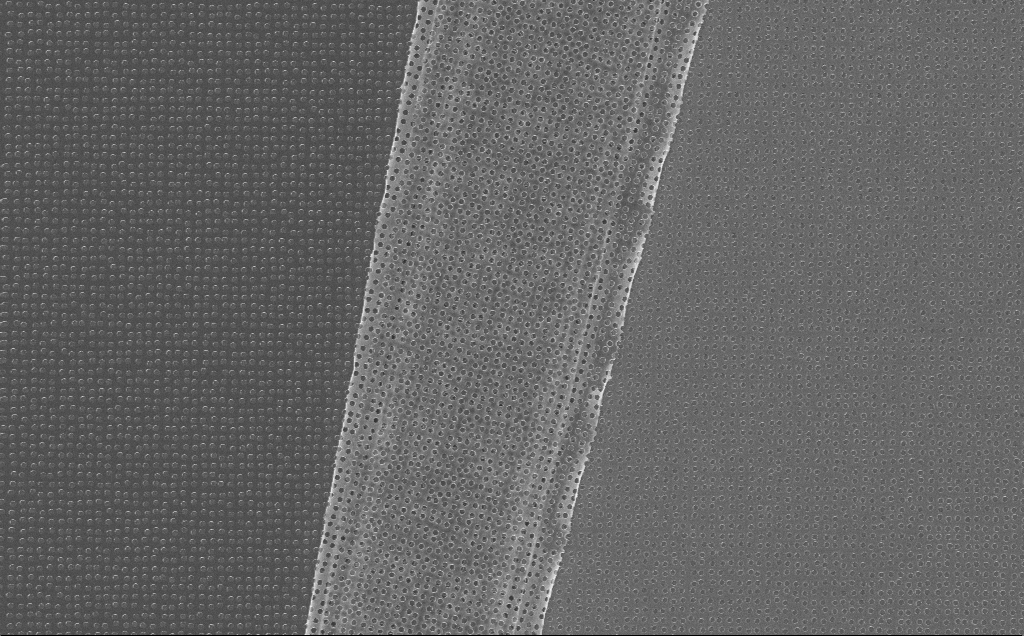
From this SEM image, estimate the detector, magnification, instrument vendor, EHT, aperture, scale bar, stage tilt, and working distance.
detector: InLens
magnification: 8.7 K X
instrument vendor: Zeiss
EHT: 10 kV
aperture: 30 µm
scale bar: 2000 nm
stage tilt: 0°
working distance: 7 mm